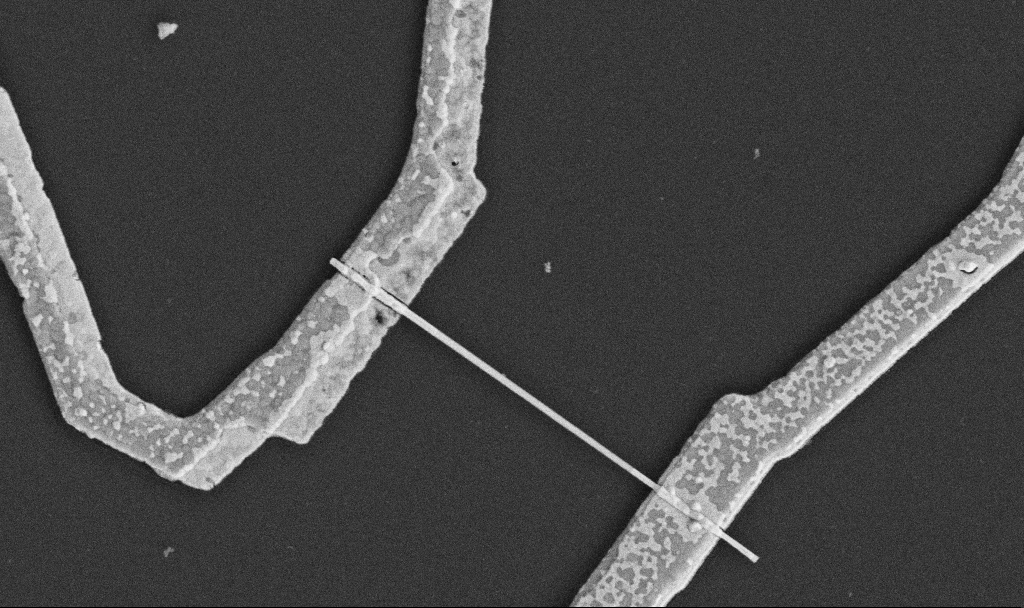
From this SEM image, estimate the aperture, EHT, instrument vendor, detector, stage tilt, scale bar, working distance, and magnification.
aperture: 30 µm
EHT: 5 kV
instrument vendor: Zeiss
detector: SE2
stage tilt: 0°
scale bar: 1000 nm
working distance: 10.6 mm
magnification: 30 K X